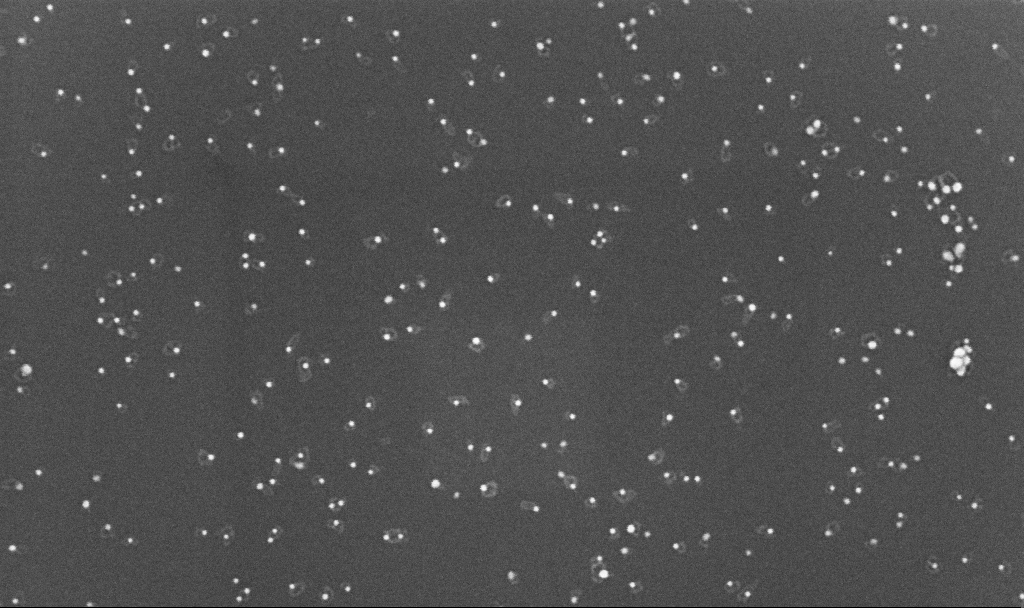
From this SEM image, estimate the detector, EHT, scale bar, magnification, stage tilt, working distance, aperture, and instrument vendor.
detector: InLens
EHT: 10 kV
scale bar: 100 nm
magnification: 200 K X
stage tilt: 0°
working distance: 3.4 mm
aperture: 30 µm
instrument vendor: Zeiss